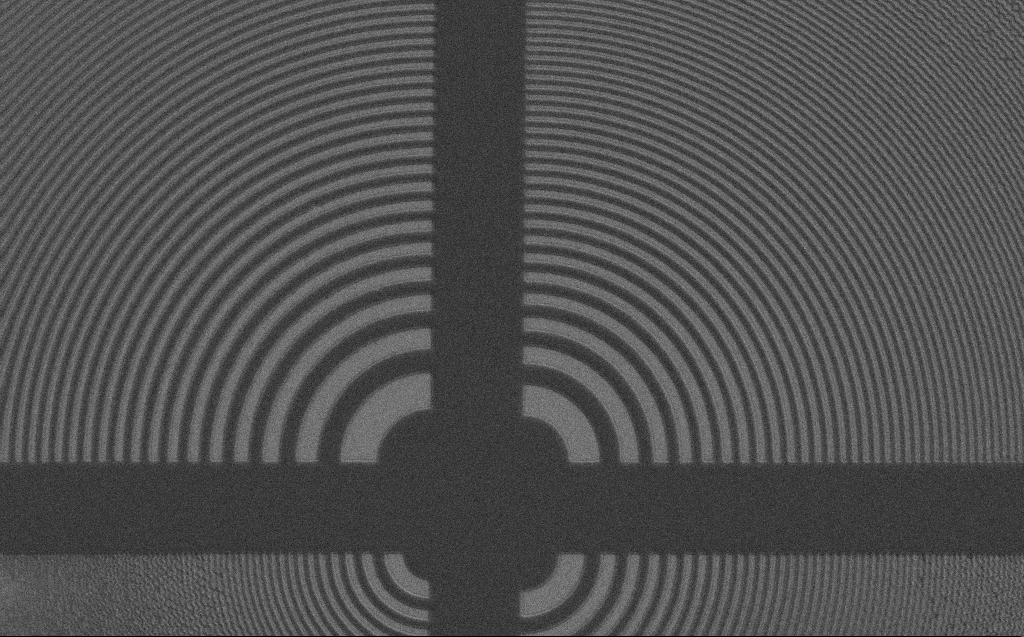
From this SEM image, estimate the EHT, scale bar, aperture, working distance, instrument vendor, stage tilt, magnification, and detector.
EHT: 3 kV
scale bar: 10000 nm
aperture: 30 µm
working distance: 4 mm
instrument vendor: Zeiss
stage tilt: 0°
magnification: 3.43 K X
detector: SE2